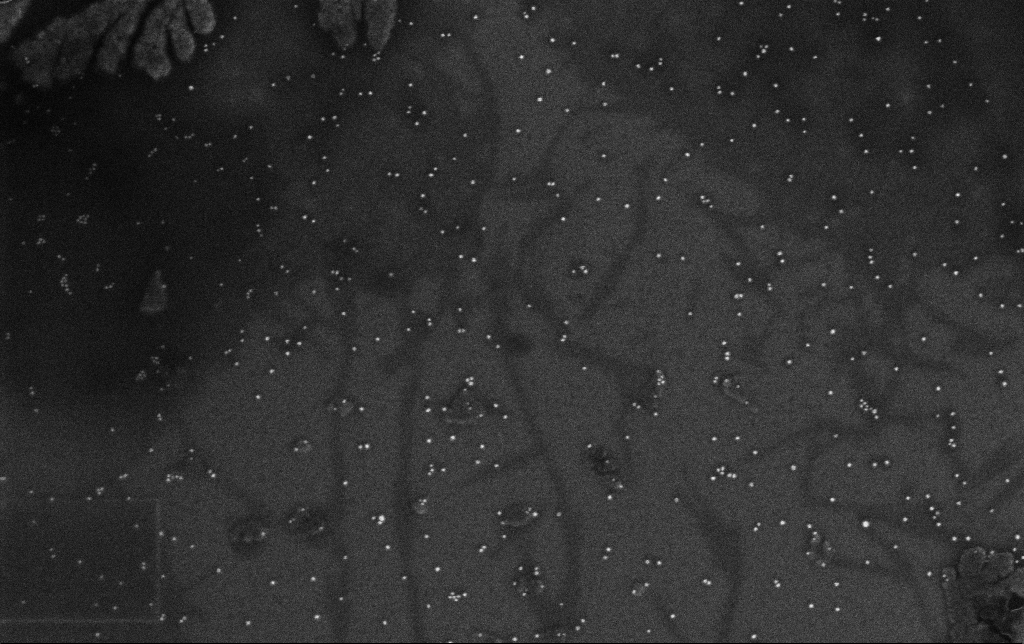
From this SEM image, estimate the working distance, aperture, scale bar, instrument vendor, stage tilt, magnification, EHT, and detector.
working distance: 3.2 mm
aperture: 30 µm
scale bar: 200 nm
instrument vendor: Zeiss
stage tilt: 0°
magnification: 100 K X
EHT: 10 kV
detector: InLens